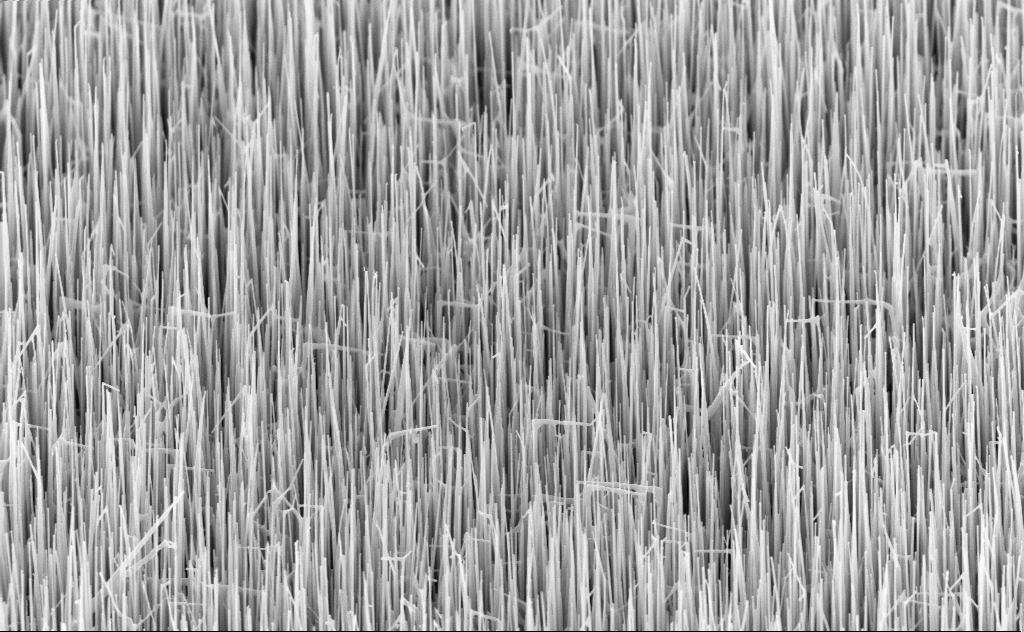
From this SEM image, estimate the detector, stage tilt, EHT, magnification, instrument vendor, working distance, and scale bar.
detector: InLens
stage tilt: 45°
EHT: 10 kV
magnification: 20 K X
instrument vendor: Zeiss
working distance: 6 mm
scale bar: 2000 nm